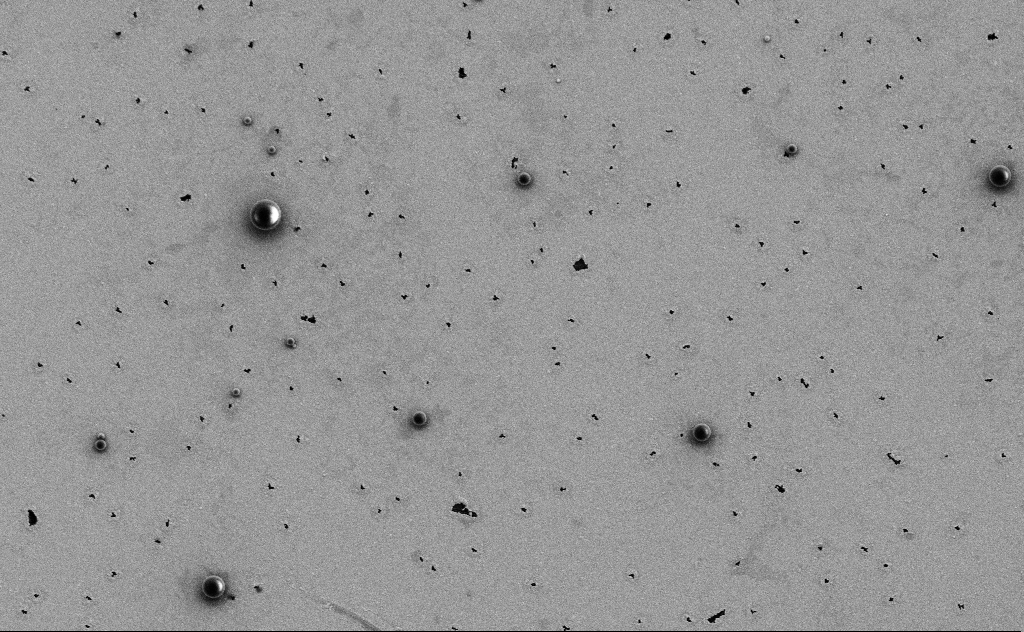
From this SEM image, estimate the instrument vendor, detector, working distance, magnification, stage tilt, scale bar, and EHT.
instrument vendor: Zeiss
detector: SE2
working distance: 10 mm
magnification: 7.27 K X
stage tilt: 0°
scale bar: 2000 nm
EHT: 3 kV